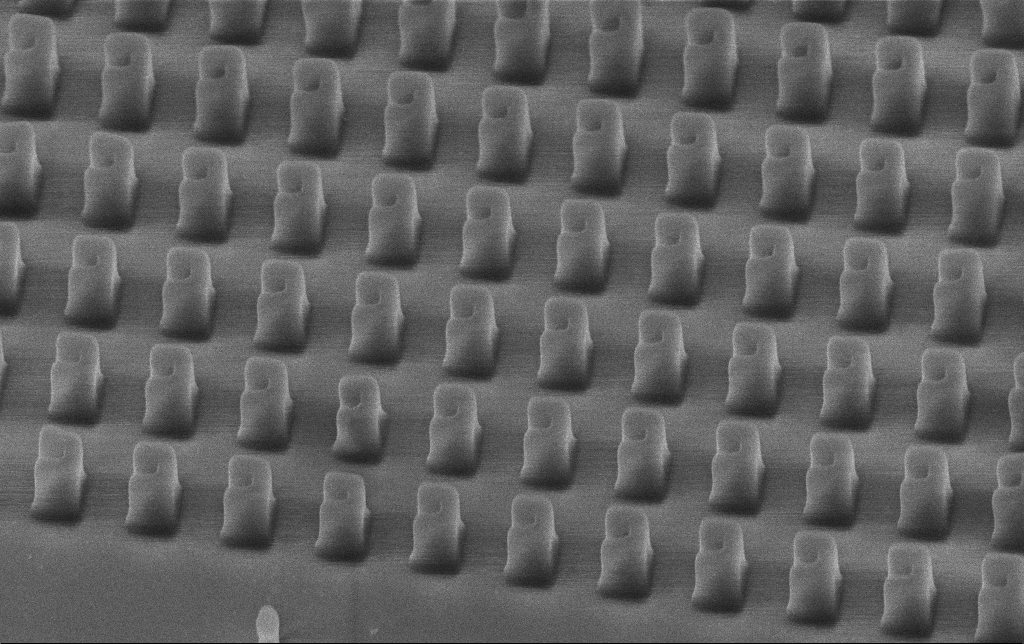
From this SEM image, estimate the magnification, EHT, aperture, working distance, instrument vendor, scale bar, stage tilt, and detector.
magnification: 35.36 K X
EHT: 3 kV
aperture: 30 µm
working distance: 7 mm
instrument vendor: Zeiss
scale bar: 1000 nm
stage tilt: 45°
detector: InLens